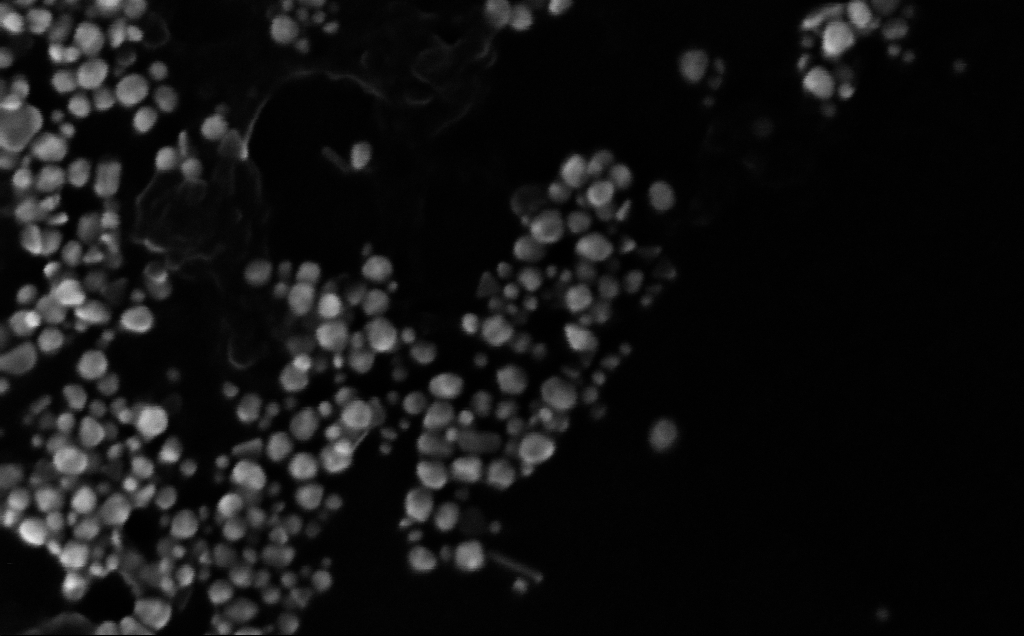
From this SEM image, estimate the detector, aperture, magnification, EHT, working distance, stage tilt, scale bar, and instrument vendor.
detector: InLens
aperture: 30 µm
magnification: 451.84 K X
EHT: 10 kV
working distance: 4 mm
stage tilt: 0°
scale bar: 100 nm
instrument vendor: Zeiss